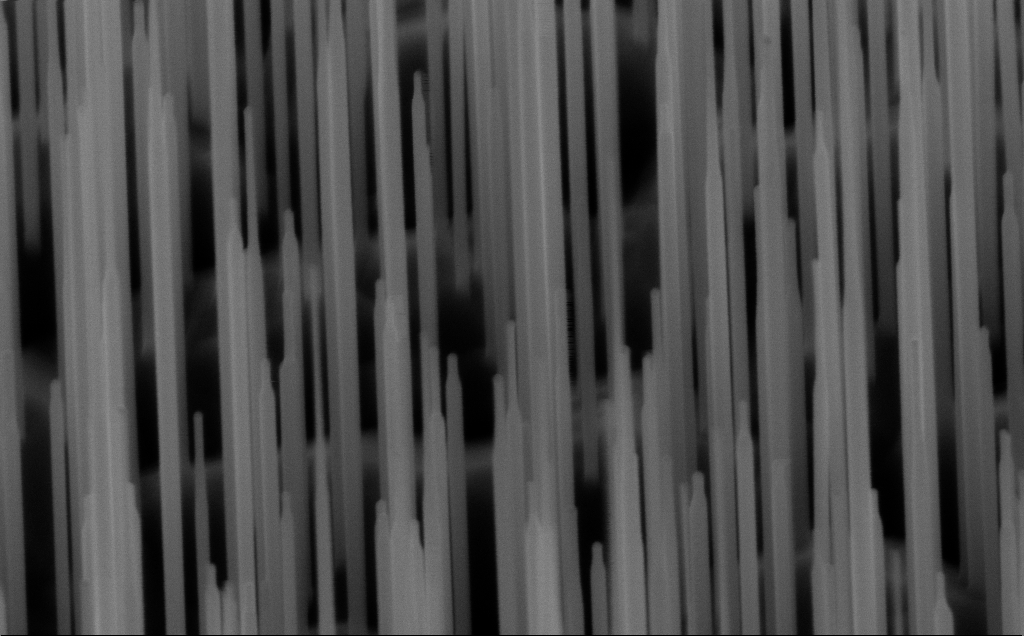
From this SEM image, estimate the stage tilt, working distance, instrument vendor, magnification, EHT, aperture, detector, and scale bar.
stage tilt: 45°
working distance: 6 mm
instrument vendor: Zeiss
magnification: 80 K X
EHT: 10 kV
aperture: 30 µm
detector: InLens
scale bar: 200 nm